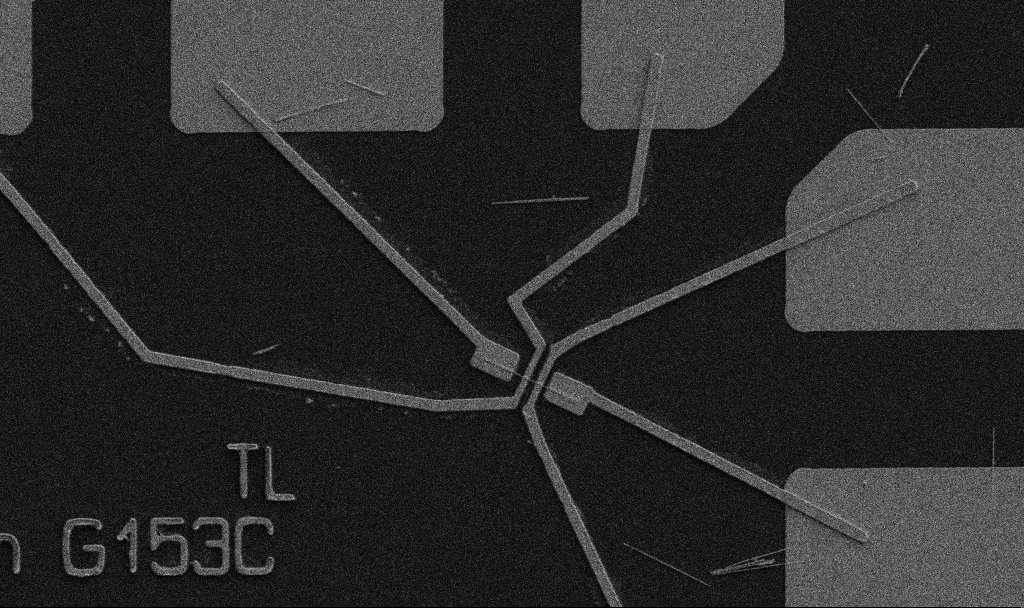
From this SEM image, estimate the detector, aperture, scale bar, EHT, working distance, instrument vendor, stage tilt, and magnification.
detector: SE2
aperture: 30 µm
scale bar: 10000 nm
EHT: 5 kV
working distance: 10.7 mm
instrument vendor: Zeiss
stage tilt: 0°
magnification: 5 K X